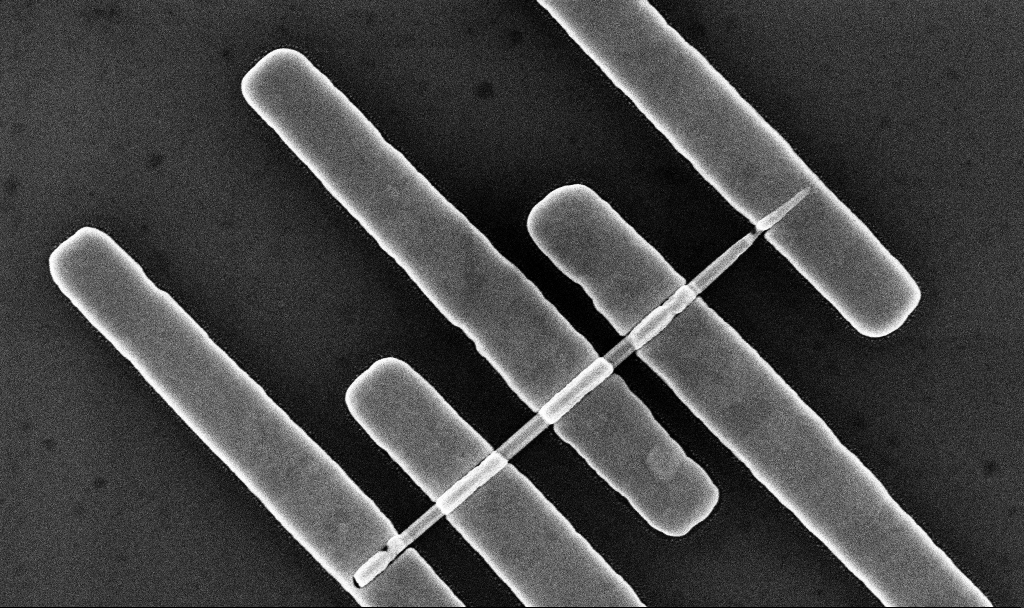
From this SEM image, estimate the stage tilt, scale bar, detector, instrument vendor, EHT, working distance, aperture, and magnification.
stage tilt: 0°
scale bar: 1000 nm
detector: InLens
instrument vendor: Zeiss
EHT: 10 kV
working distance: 7.7 mm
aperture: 30 µm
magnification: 45.1 K X